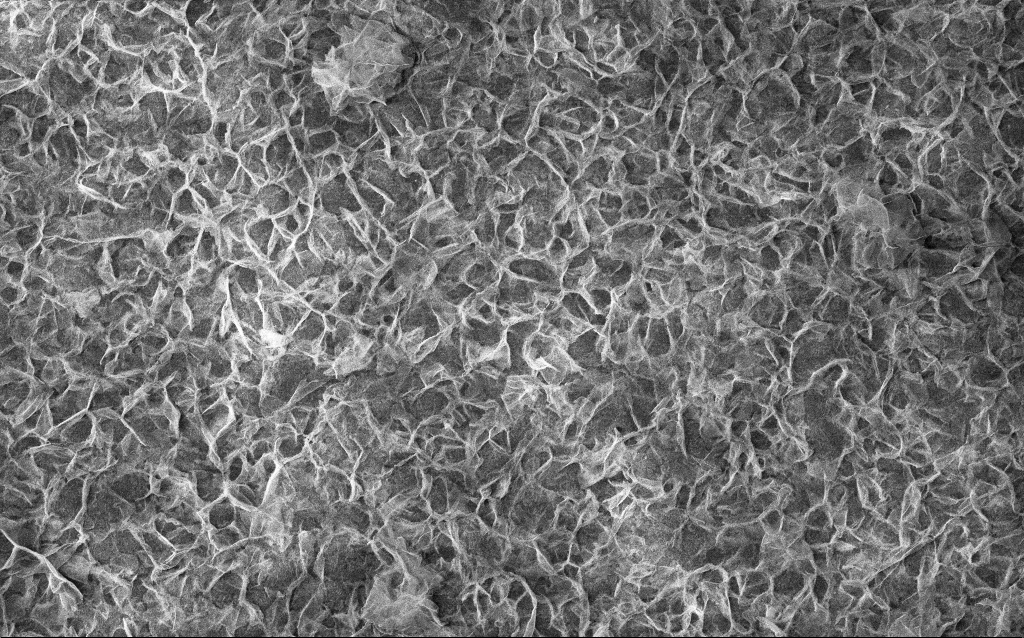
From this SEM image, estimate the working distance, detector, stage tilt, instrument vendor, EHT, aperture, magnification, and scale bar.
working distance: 2.8 mm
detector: InLens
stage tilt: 0°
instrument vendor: Zeiss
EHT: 10 kV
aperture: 30 µm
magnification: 10 K X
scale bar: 2000 nm